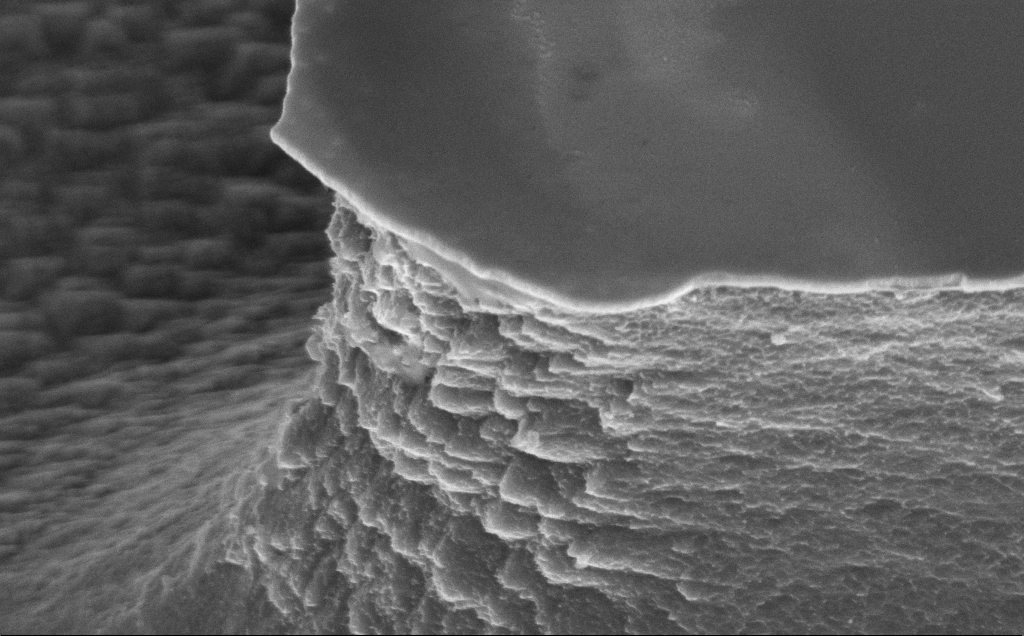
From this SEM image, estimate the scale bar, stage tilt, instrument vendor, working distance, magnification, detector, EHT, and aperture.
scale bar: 2000 nm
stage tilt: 45°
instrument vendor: Zeiss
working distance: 13 mm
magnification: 35.12 K X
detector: InLens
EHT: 5 kV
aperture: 30 µm